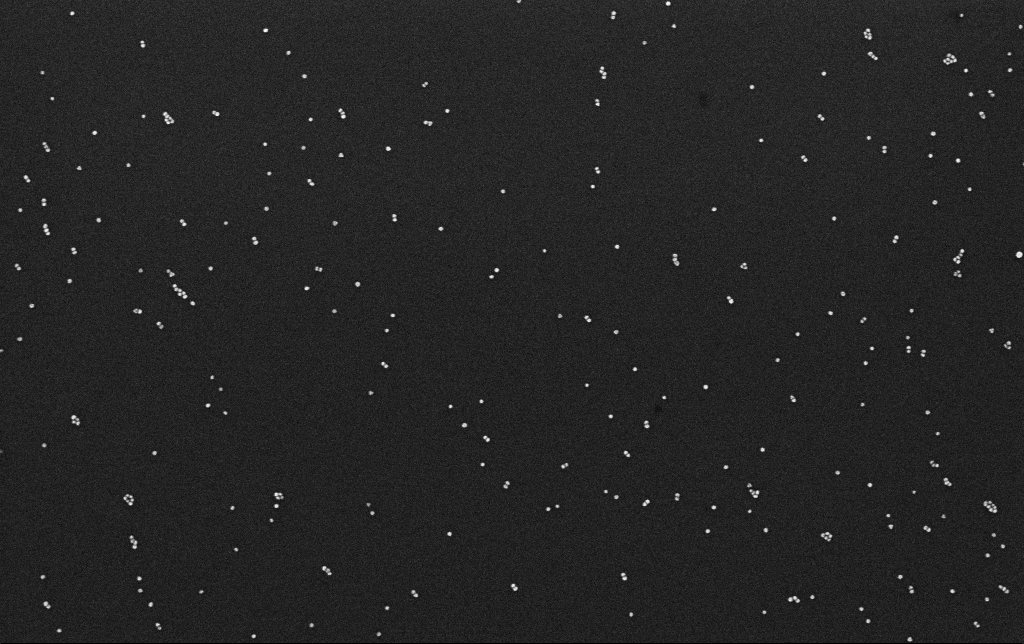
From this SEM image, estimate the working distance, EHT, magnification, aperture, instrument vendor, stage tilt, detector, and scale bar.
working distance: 3.1 mm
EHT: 10 kV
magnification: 100 K X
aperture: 30 µm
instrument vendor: Zeiss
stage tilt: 0°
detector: InLens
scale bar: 200 nm